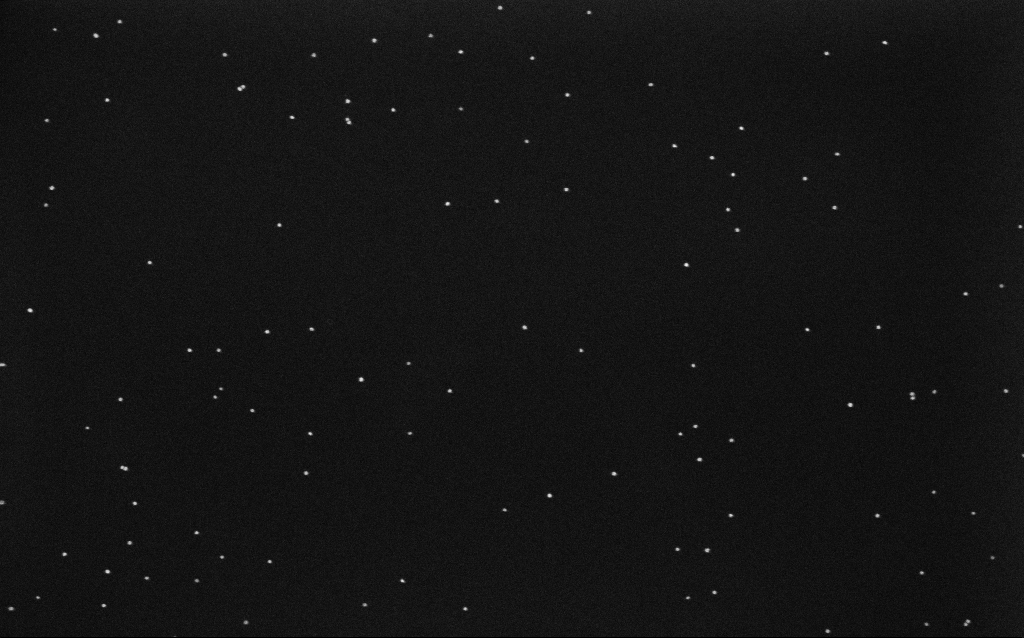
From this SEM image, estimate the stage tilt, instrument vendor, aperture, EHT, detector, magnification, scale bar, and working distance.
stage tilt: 0°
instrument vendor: Zeiss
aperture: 30 µm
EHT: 10 kV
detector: InLens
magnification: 100 K X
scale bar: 200 nm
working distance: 6.5 mm